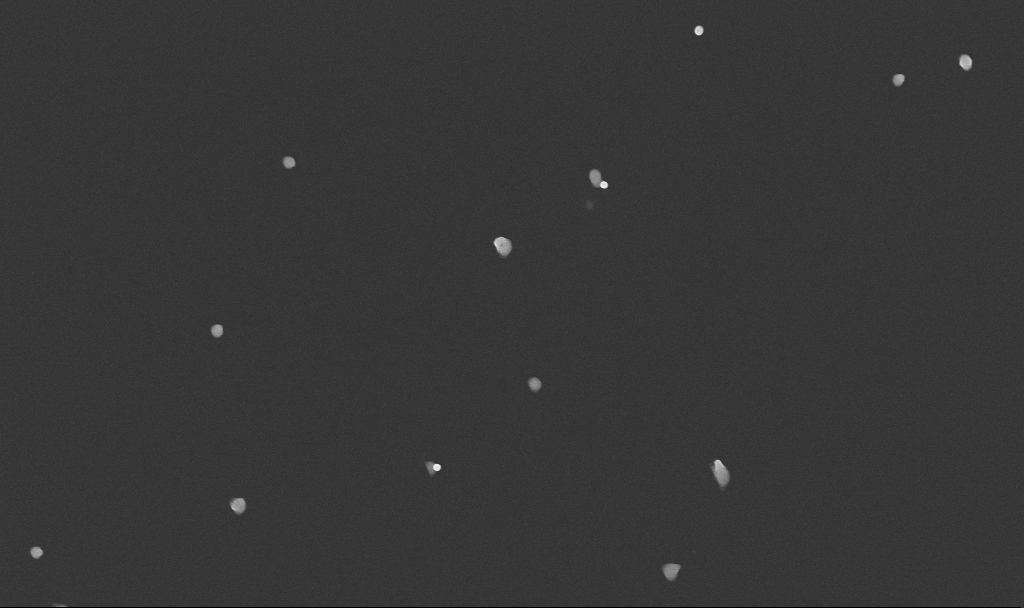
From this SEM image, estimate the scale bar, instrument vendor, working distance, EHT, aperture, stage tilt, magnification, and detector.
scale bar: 1000 nm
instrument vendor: Zeiss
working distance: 3.4 mm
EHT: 10 kV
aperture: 30 µm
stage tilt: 0°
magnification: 50 K X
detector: InLens